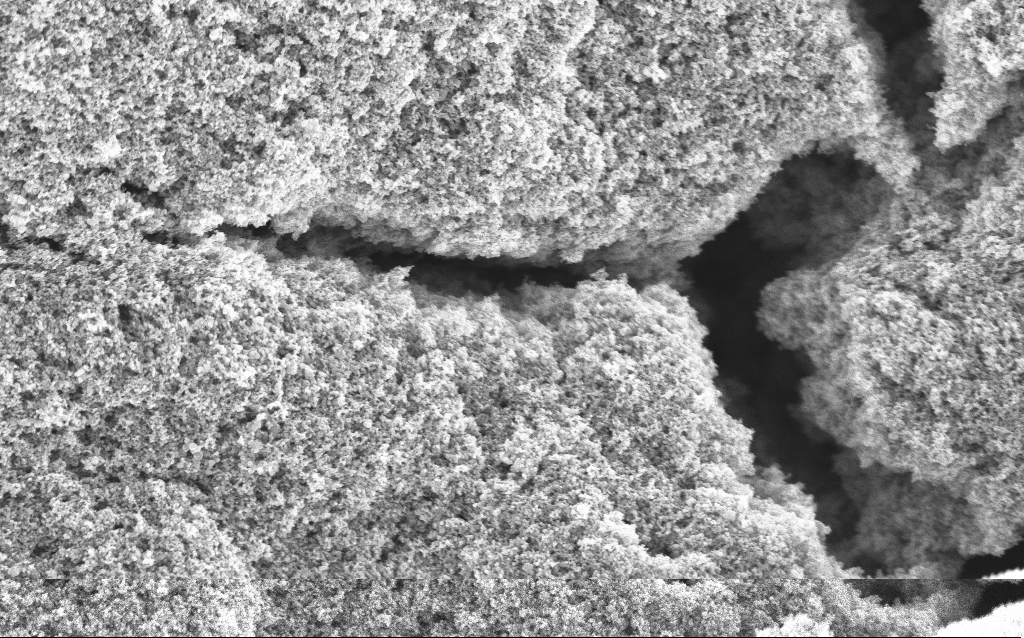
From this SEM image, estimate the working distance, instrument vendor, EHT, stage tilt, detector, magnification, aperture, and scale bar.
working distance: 4.2 mm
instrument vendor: Zeiss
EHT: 5 kV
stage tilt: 0°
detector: InLens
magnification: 37.88 K X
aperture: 30 µm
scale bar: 1000 nm